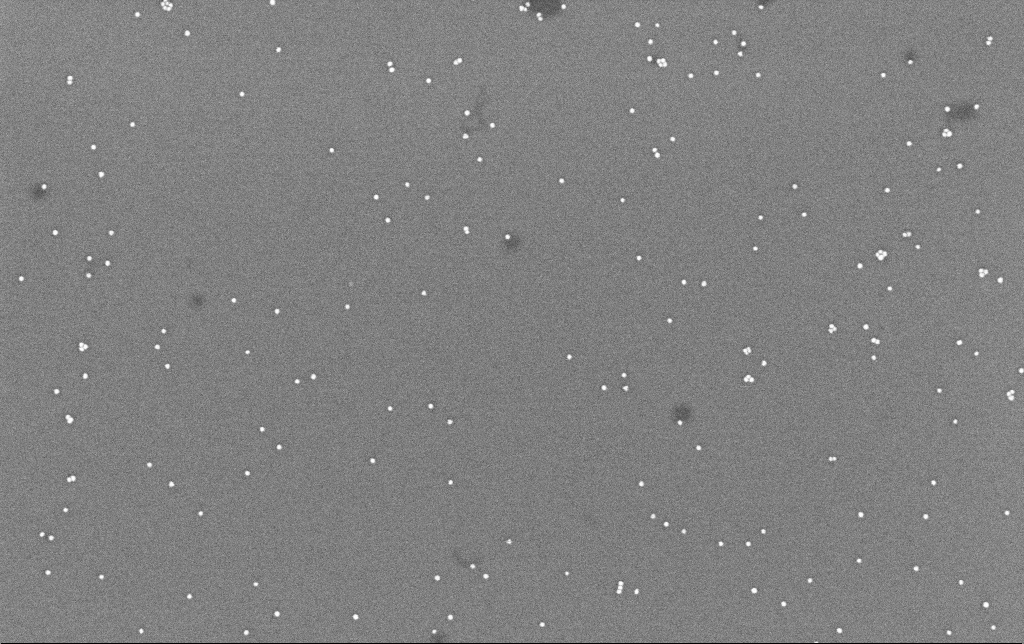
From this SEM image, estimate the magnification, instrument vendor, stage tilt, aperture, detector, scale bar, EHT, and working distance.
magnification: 100 K X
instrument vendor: Zeiss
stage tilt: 0°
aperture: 30 µm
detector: InLens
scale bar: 200 nm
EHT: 8 kV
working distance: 6.6 mm